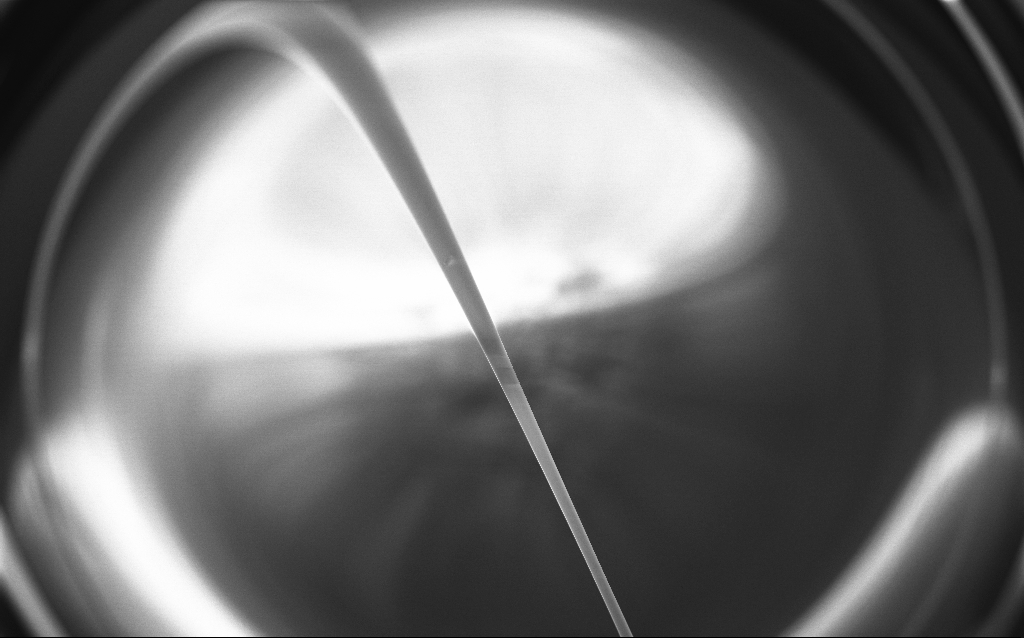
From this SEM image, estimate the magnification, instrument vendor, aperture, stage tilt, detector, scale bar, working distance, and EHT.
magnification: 0.1 K X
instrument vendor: Zeiss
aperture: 30 µm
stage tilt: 45°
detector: InLens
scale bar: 200000 nm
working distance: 7 mm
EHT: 1 kV